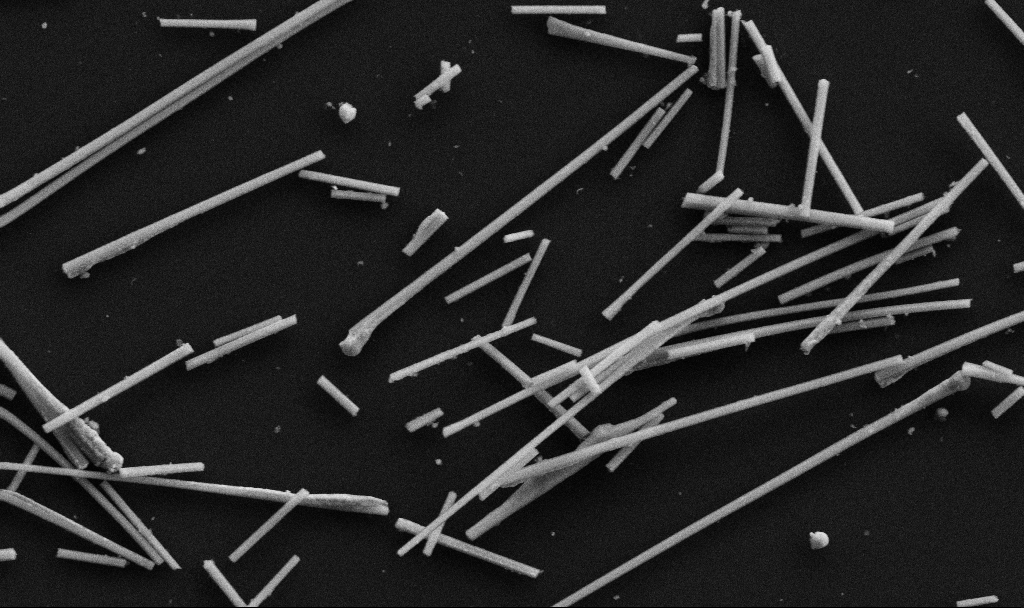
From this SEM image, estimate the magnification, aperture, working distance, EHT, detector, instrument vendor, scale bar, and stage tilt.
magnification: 35.19 K X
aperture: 30 µm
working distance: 6.7 mm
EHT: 5 kV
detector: SE2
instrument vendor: Zeiss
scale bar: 1000 nm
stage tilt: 0°